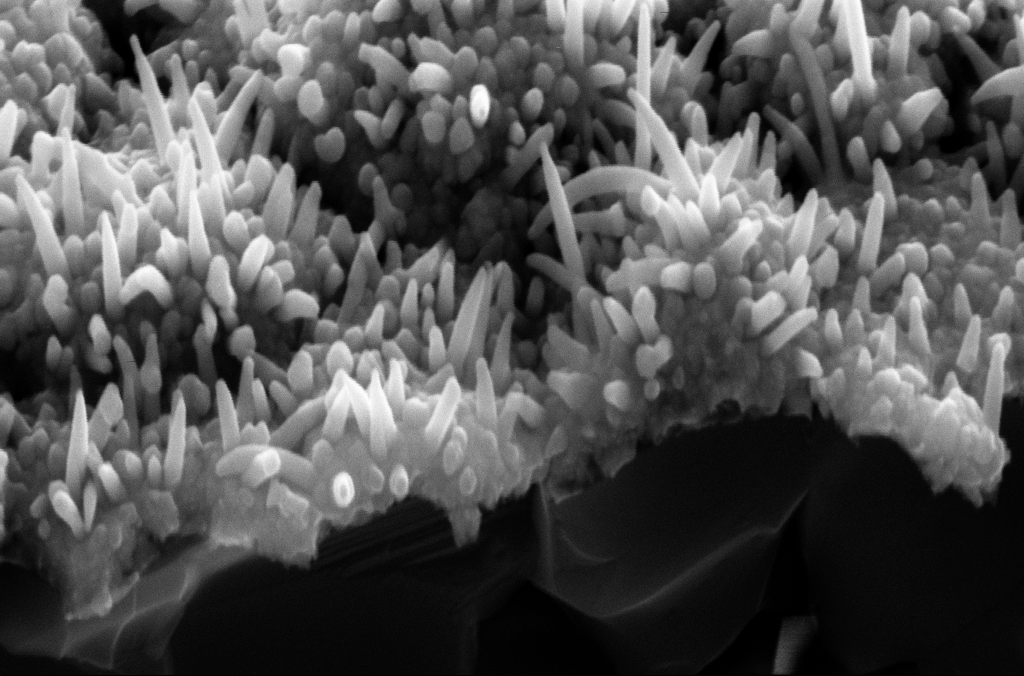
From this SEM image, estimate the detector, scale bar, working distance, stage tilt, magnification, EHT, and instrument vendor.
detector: SE2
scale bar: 200 nm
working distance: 8 mm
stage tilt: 45°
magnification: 106.23 K X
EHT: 10 kV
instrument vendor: Zeiss